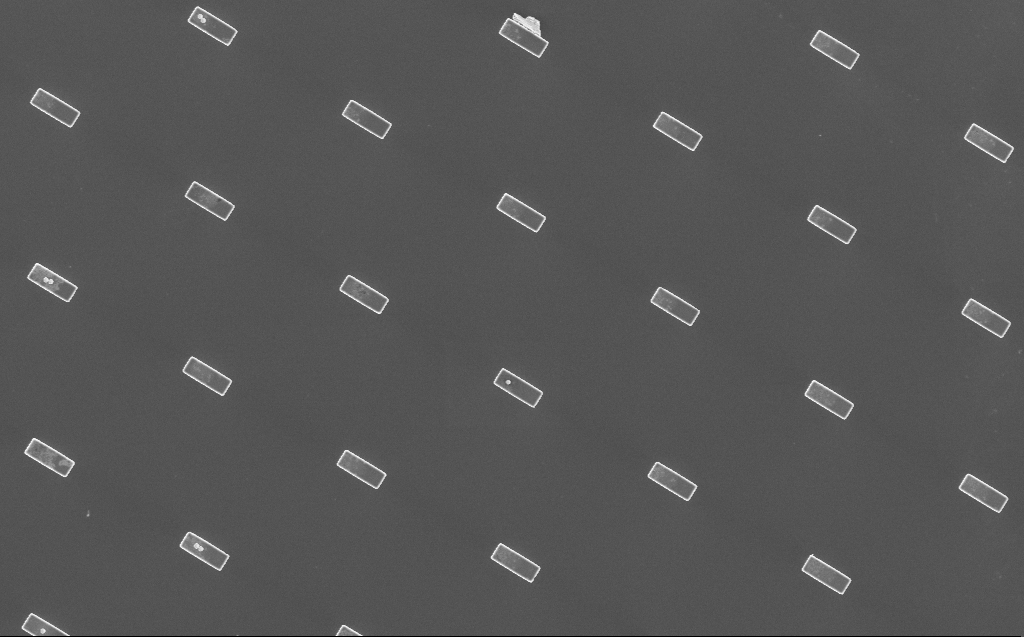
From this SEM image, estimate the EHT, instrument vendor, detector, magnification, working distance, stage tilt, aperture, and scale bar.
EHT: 5 kV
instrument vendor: Zeiss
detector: InLens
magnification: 2.33 K X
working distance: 8 mm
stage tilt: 0°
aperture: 30 µm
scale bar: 10000 nm